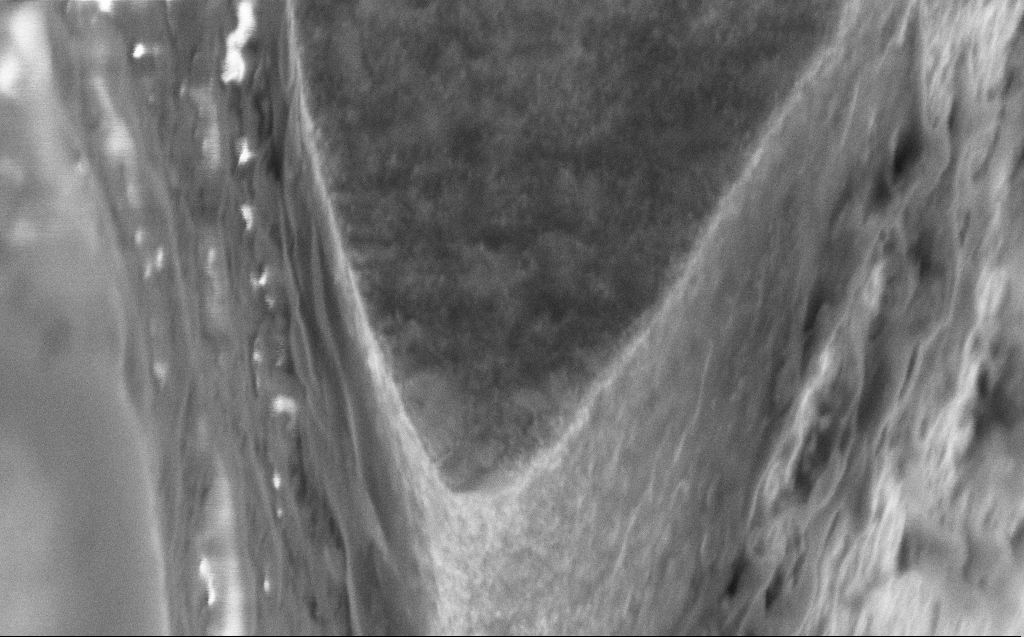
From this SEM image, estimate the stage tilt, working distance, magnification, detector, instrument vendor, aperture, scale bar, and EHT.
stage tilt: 45°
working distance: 7 mm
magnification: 70.38 K X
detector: InLens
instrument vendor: Zeiss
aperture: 30 µm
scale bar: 1000 nm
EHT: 3 kV